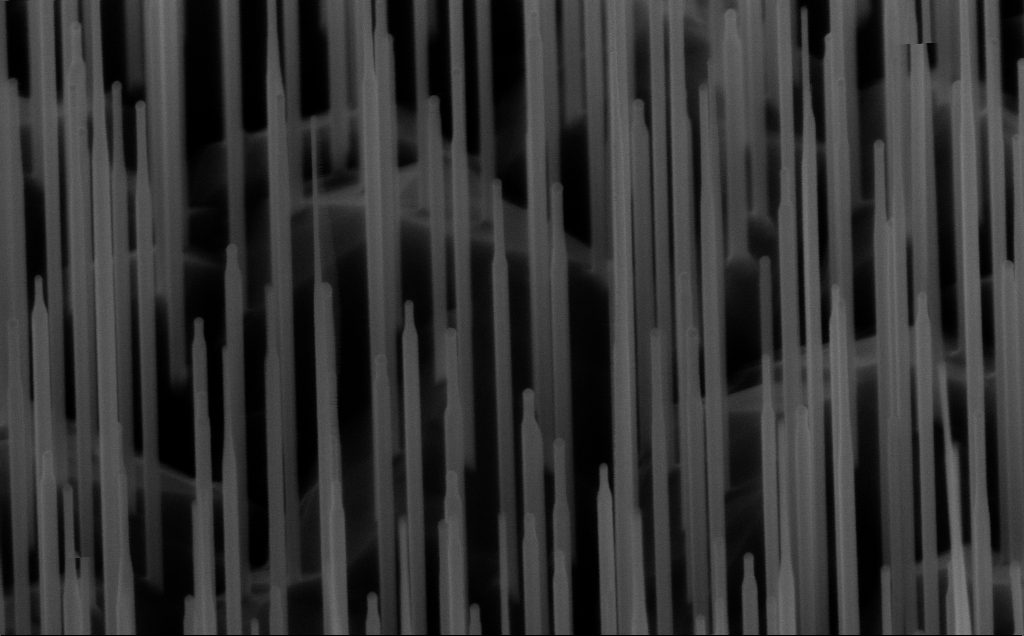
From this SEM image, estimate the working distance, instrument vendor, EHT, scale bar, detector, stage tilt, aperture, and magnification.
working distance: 6 mm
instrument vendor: Zeiss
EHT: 10 kV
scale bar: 200 nm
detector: InLens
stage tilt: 45°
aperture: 30 µm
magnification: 80 K X